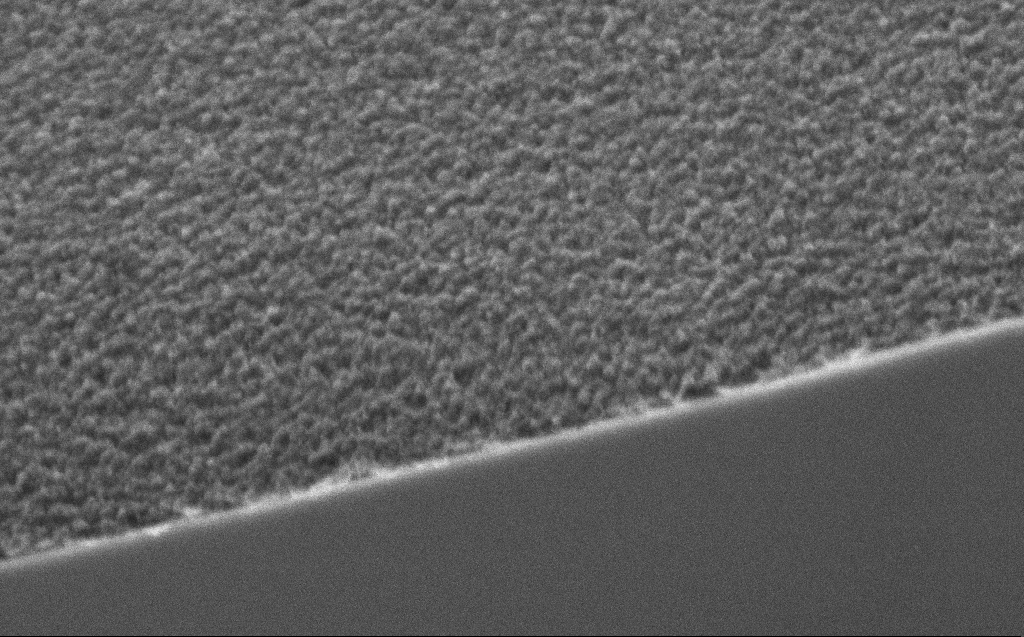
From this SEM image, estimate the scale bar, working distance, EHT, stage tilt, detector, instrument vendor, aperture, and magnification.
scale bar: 200 nm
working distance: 6 mm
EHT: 1.5 kV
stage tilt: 44.9°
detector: InLens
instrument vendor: Zeiss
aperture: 20 µm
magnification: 84.51 K X